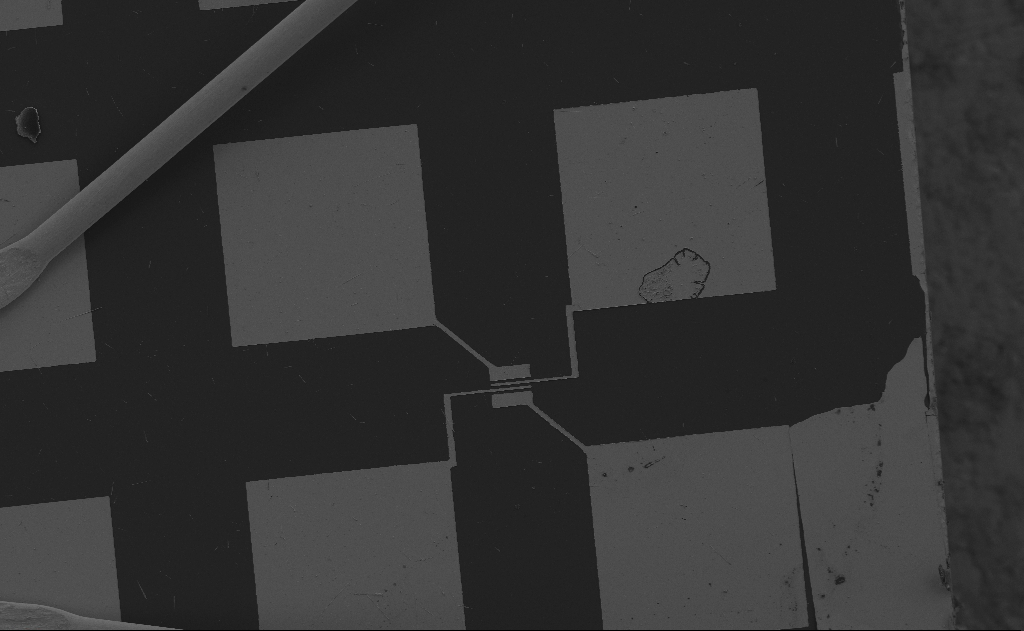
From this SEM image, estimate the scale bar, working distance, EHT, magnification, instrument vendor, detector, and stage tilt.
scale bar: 100000 nm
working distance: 12 mm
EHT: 5 kV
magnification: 0.501 K X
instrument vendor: Zeiss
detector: SE2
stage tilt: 0°